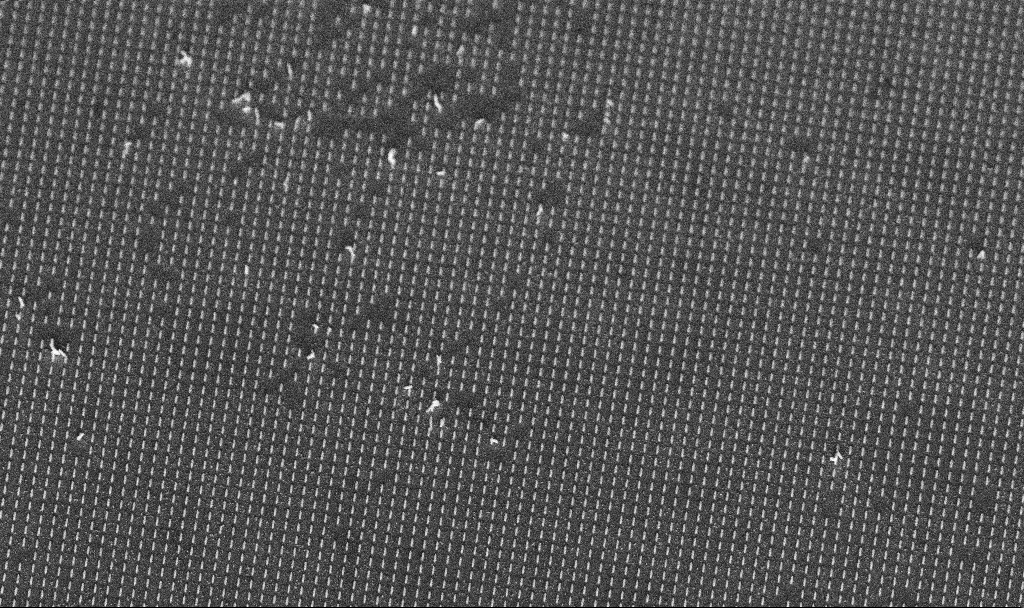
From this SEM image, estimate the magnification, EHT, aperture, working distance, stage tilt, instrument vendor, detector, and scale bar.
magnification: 20.3 K X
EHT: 5 kV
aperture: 30 µm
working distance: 7.4 mm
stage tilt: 45°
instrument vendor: Zeiss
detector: InLens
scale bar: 1000 nm